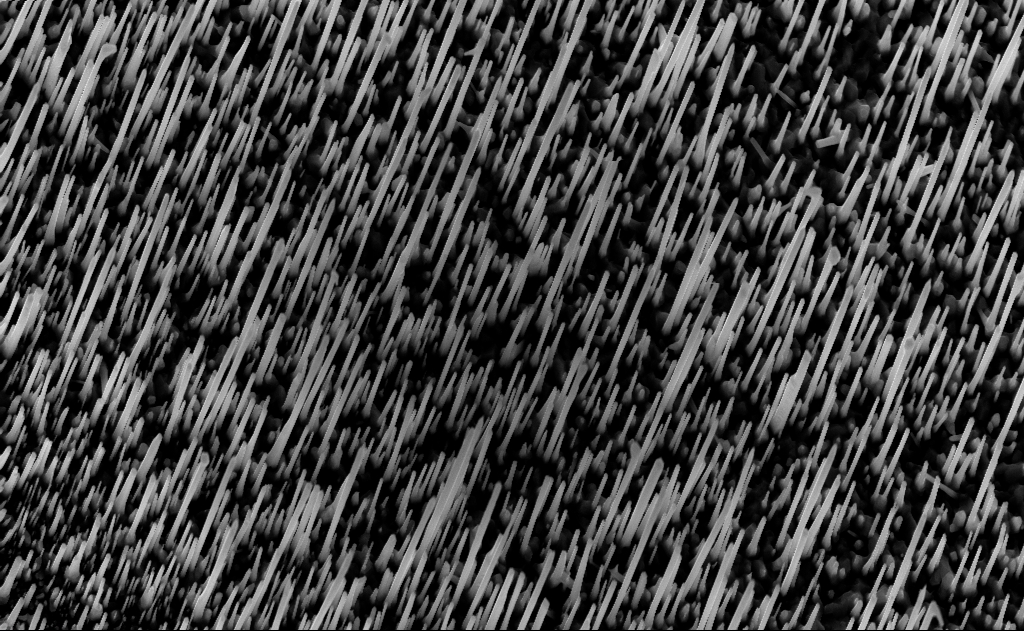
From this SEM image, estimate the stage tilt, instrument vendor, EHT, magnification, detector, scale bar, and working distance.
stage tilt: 0°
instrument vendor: Zeiss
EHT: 10 kV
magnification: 20 K X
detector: InLens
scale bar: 1000 nm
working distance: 7 mm